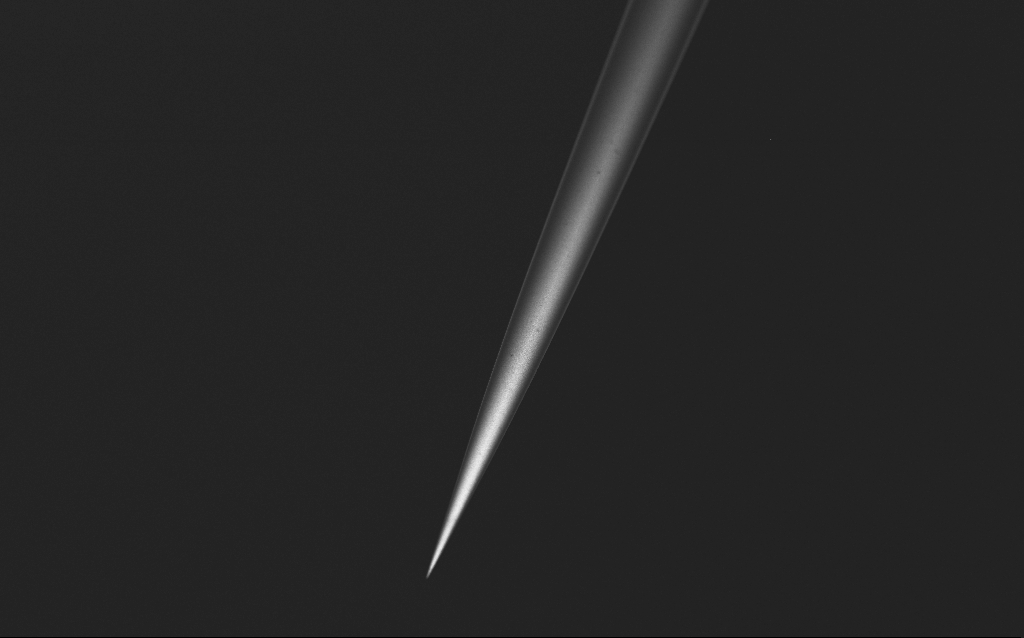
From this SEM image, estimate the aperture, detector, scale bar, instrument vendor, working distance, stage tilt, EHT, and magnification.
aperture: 30 µm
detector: InLens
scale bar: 20000 nm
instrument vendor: Zeiss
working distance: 5 mm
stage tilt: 45°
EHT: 2.5 kV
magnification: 1 K X